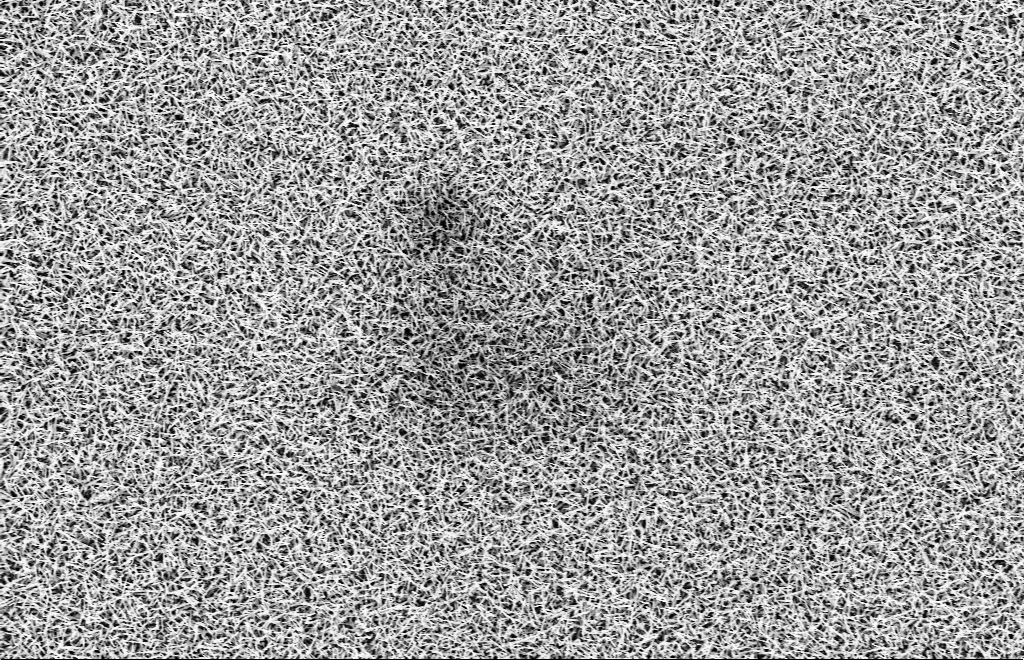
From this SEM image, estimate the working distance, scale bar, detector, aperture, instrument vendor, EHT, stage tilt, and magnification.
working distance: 15 mm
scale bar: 2000 nm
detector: InLens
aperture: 30 µm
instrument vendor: Zeiss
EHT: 10 kV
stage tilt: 0°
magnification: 5 K X